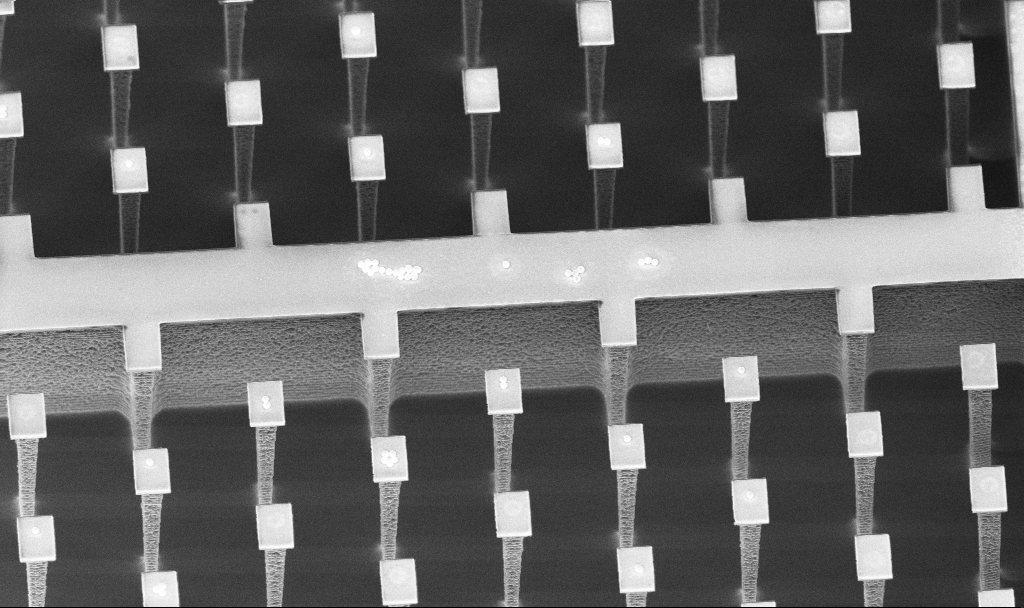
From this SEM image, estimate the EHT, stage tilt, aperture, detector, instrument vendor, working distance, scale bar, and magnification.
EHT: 5 kV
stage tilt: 30°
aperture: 30 µm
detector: InLens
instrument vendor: Zeiss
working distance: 4.5 mm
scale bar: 10000 nm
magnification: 4.38 K X